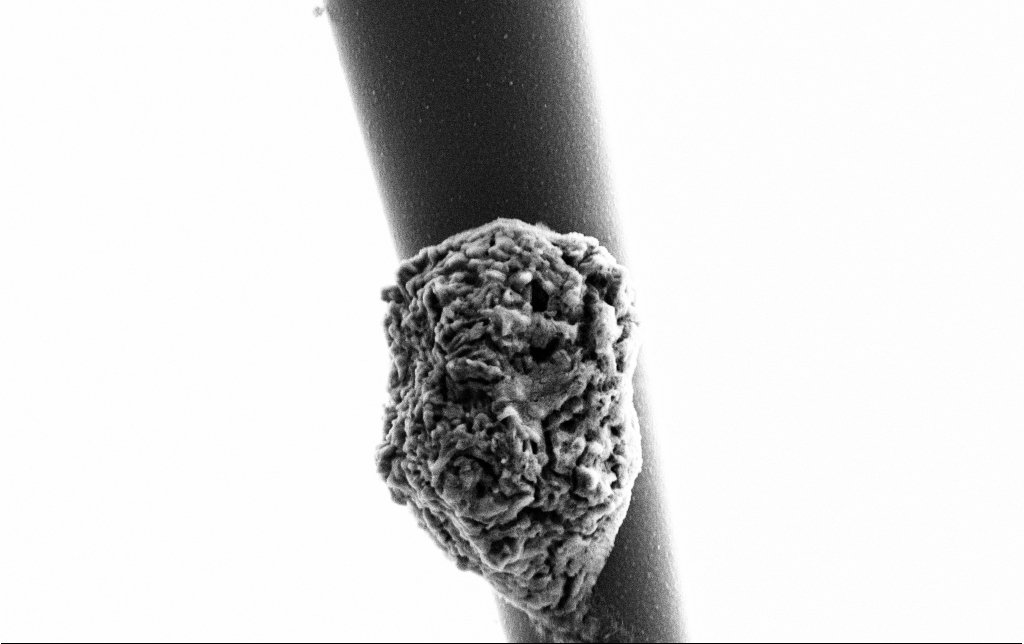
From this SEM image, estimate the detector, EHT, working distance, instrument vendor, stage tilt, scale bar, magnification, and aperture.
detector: SE2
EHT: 3 kV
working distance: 6.5 mm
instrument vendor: Zeiss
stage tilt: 0°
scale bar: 2000 nm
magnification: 15 K X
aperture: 30 µm